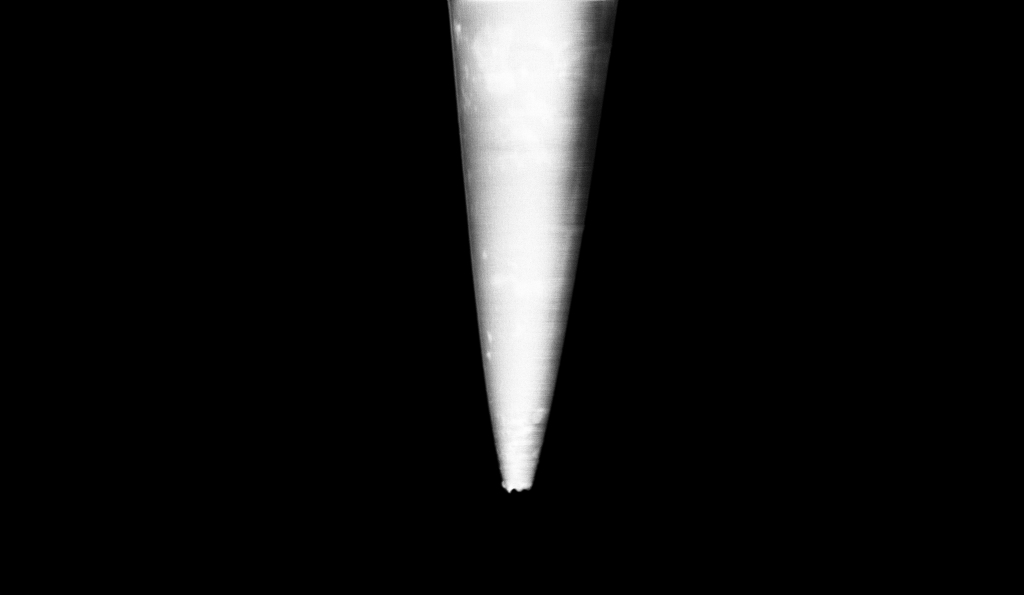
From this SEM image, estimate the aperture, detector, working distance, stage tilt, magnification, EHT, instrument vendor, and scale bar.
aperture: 30 µm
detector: InLens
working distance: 6.8 mm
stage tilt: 0°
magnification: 5 K X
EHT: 1 kV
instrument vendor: Zeiss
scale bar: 10000 nm